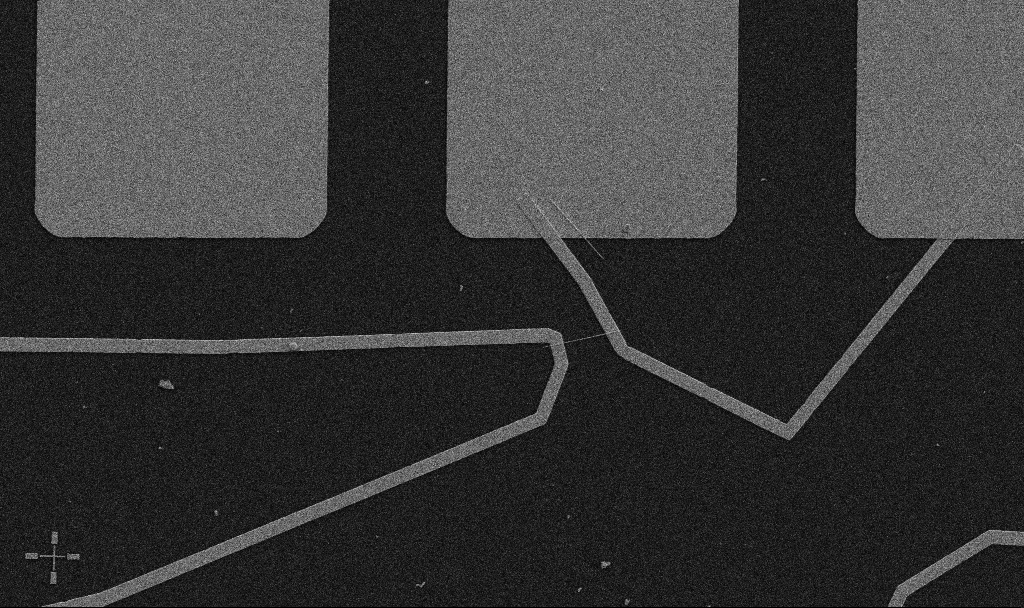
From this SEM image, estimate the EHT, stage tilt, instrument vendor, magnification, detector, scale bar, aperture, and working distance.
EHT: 5 kV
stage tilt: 0°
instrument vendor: Zeiss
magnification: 5 K X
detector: SE2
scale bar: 10000 nm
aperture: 30 µm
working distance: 10.7 mm